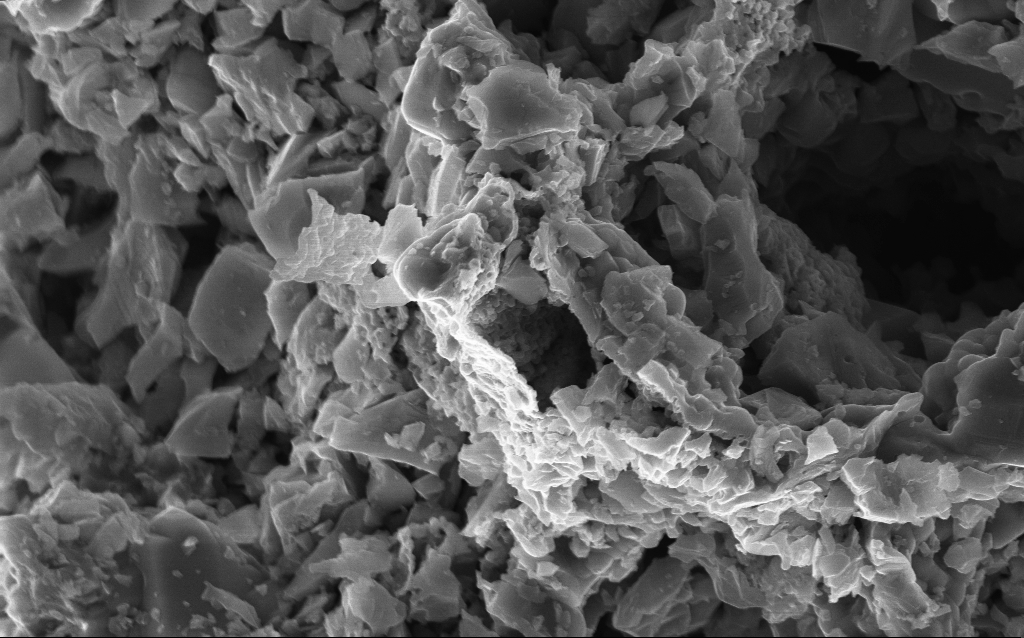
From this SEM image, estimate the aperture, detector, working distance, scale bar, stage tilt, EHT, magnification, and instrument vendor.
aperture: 30 µm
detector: InLens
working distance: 3 mm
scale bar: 2000 nm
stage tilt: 0°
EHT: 10 kV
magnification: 15 K X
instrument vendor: Zeiss